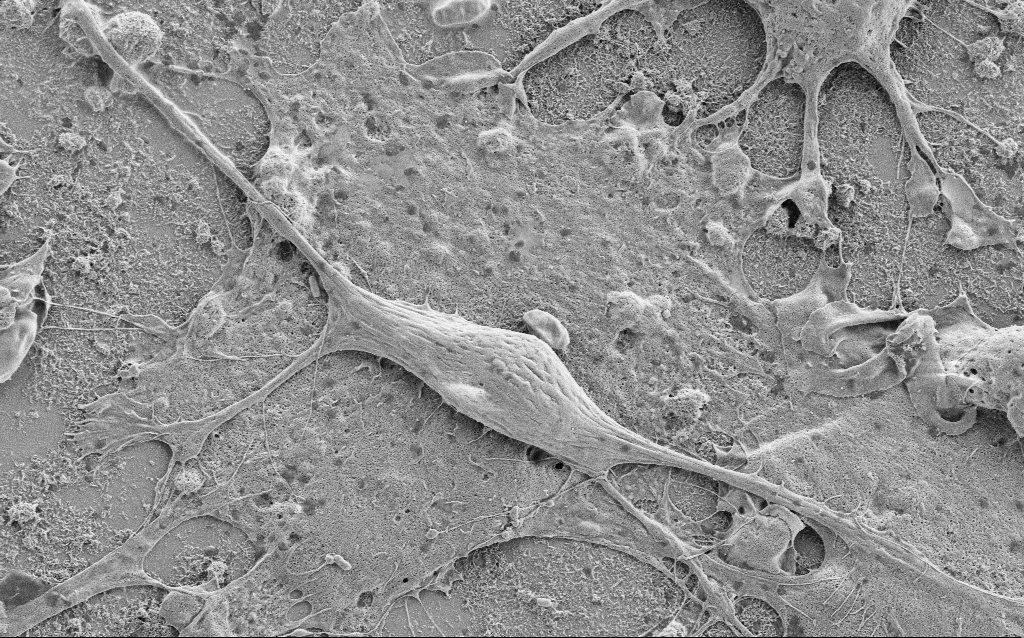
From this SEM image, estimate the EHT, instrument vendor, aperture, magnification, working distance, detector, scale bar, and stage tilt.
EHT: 2 kV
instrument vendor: Zeiss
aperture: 30 µm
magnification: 5 K X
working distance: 6.9 mm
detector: SE2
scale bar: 10000 nm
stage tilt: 0°